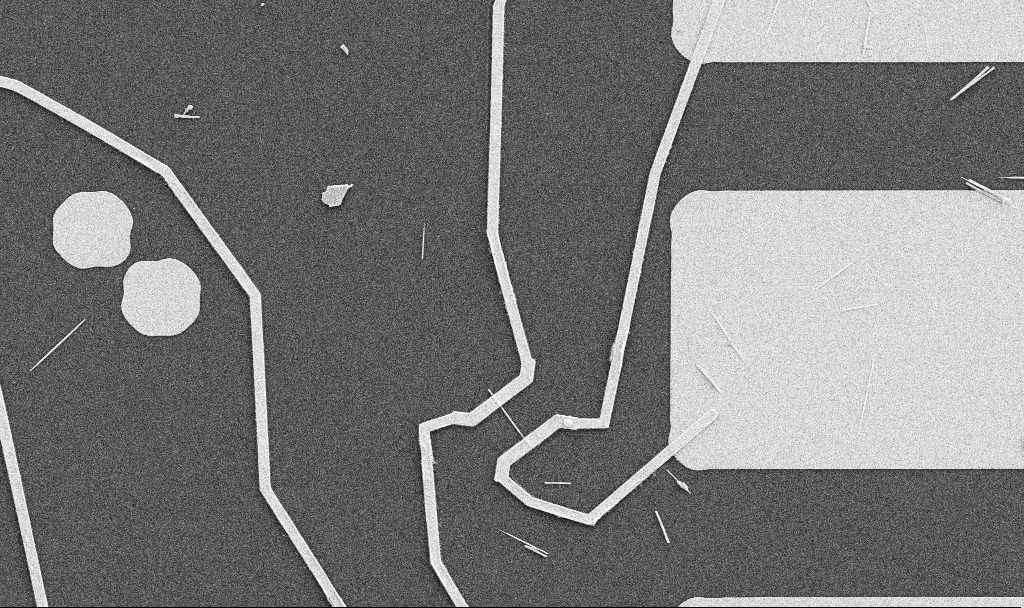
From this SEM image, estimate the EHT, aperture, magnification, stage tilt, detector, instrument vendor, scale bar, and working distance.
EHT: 5 kV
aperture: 30 µm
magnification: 5 K X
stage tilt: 0°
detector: SE2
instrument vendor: Zeiss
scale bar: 10000 nm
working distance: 10.7 mm